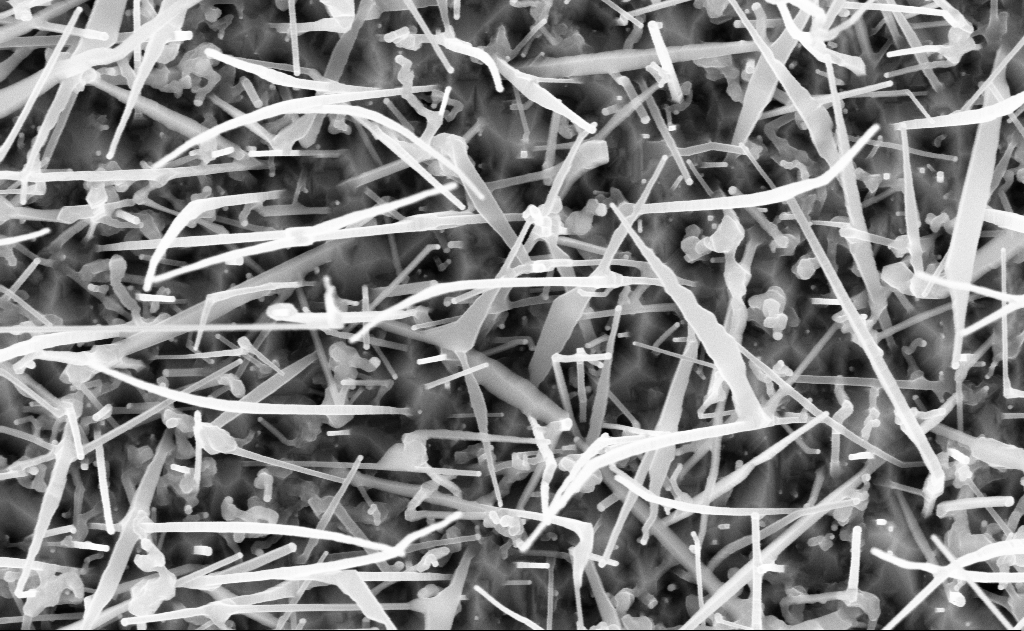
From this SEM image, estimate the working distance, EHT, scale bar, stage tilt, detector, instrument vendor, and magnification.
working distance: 11 mm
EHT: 10 kV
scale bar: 1000 nm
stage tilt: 0°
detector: InLens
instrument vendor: Zeiss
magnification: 60 K X